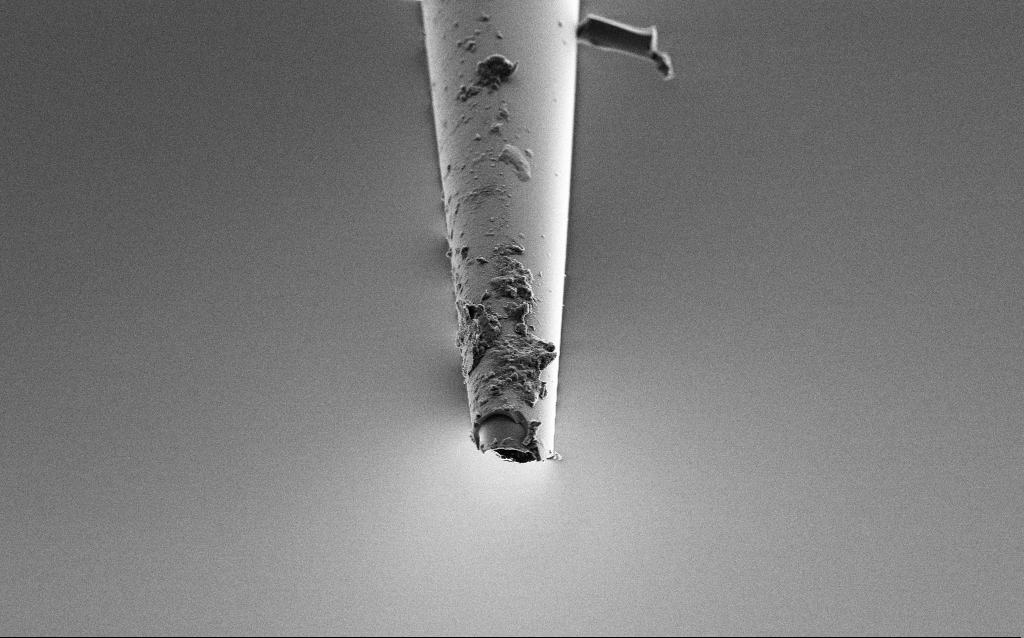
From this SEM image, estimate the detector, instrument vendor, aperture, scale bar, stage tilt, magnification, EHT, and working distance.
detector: SE2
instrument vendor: Zeiss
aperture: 30 µm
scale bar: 10000 nm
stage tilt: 45°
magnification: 5 K X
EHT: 2 kV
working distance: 6 mm